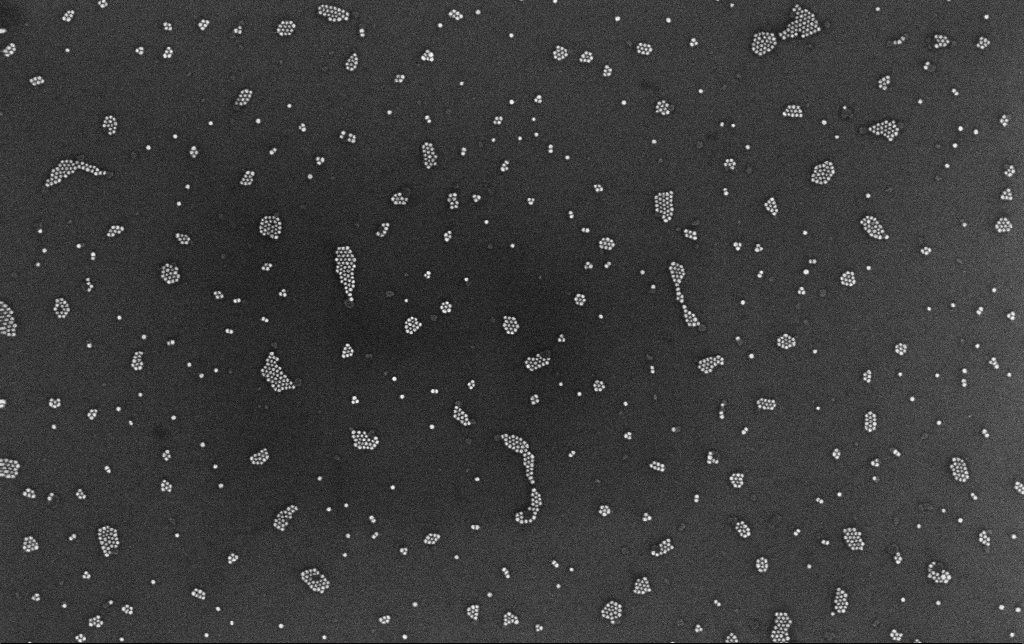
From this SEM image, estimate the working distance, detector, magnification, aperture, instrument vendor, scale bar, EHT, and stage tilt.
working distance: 3.4 mm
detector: InLens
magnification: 100 K X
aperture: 30 µm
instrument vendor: Zeiss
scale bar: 200 nm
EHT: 10 kV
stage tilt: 0°